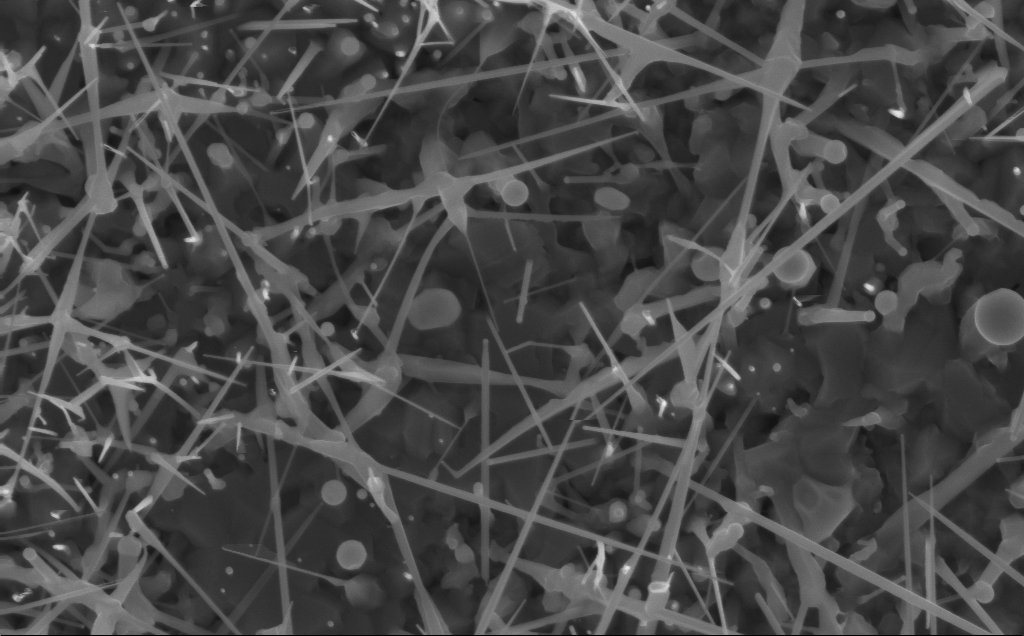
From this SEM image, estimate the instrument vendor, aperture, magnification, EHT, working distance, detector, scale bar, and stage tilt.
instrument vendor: Zeiss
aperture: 30 µm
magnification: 40 K X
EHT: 10 kV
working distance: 3 mm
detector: InLens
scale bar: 1000 nm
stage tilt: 0°